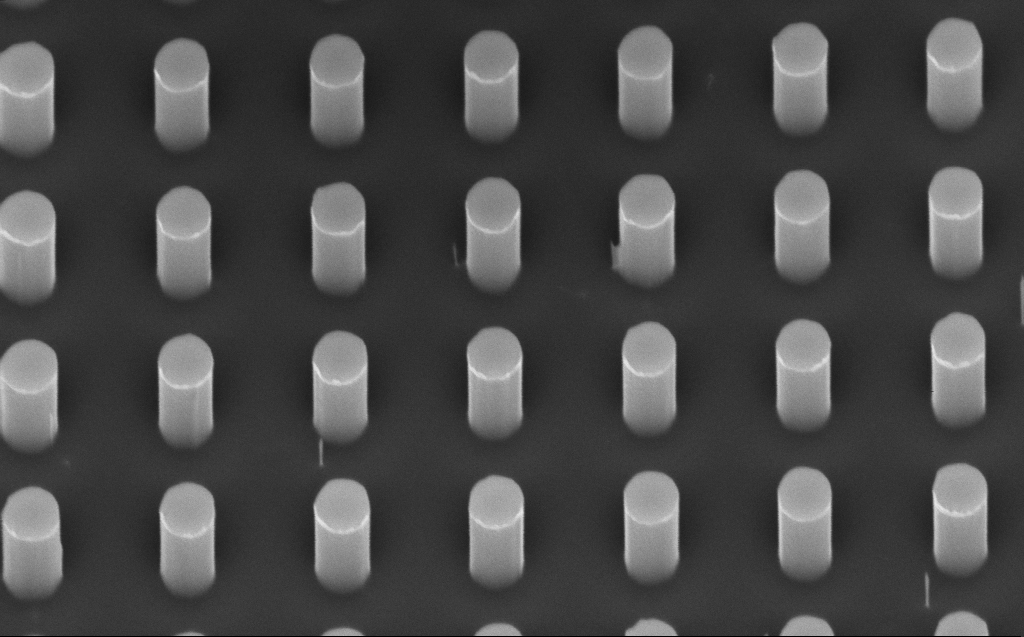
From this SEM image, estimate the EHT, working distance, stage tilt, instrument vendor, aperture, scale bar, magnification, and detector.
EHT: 5 kV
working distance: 6 mm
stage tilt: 45°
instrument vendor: Zeiss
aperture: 30 µm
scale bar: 1000 nm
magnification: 54.26 K X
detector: InLens